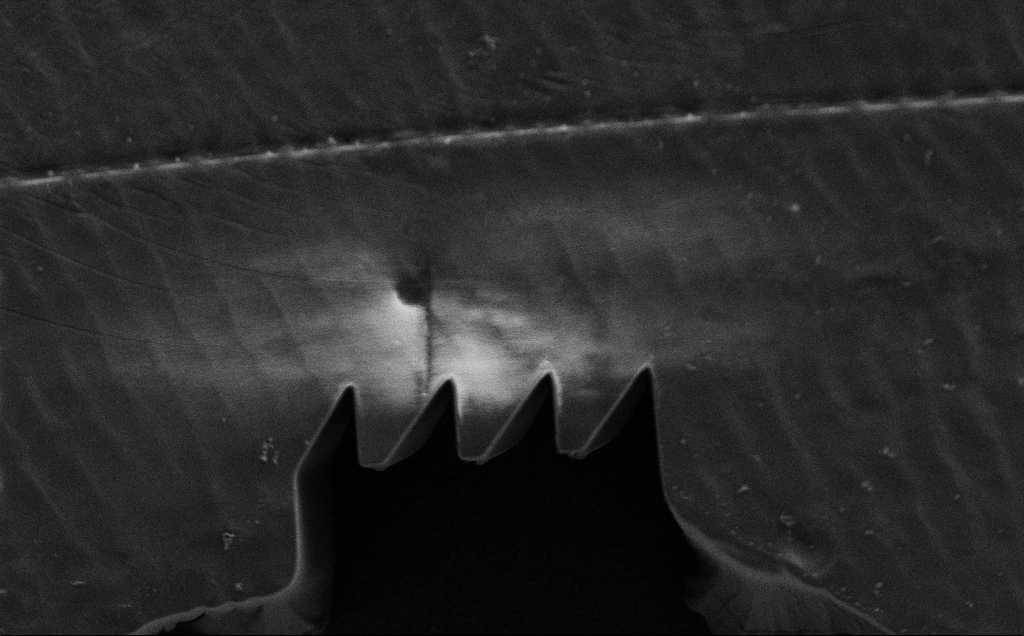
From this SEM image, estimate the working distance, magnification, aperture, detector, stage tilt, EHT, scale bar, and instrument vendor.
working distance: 8 mm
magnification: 3.14 K X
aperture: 30 µm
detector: SE2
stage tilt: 0°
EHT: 1.2 kV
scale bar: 20000 nm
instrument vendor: Zeiss